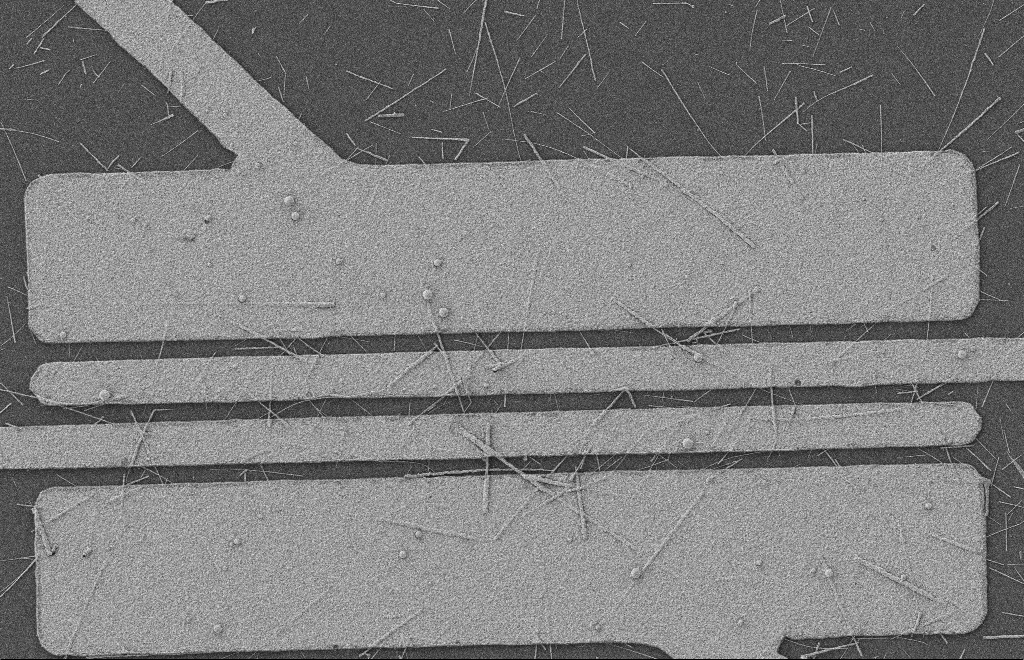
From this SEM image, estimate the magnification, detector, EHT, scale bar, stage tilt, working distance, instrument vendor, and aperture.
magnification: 5.71 K X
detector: SE2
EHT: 2 kV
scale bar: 2000 nm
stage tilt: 0°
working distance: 8 mm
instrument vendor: Zeiss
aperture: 20 µm